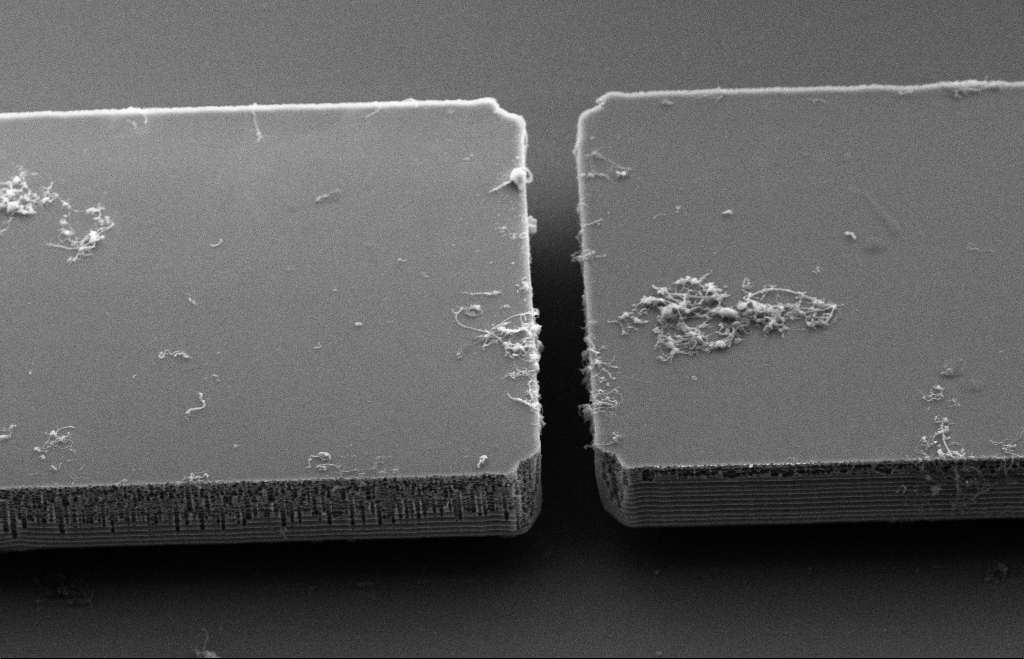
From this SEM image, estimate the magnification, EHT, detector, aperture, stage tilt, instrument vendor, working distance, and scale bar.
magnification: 9.91 K X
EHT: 5 kV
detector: SE2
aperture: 20 µm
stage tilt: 39°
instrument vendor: Zeiss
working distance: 9 mm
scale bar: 2000 nm